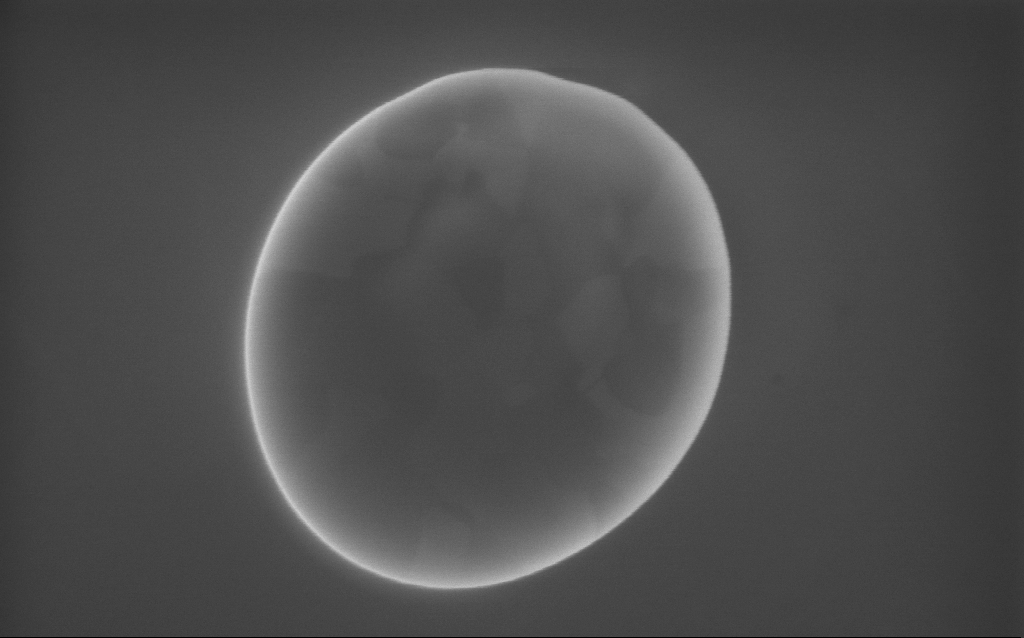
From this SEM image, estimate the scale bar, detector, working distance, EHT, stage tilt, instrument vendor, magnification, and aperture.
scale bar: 200 nm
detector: InLens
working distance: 3 mm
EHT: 10 kV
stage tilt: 0°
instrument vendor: Zeiss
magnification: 97 K X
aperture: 30 µm